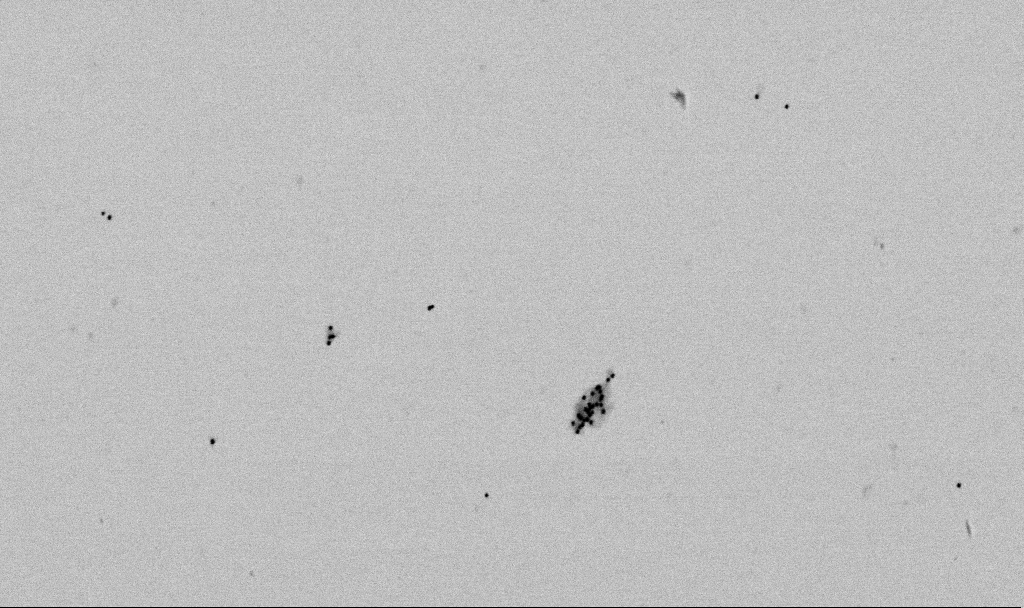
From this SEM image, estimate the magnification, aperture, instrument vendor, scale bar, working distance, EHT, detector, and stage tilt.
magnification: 50 K X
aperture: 30 µm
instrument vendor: Zeiss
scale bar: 1000 nm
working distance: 6.5 mm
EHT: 2 kV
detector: SE2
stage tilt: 0°